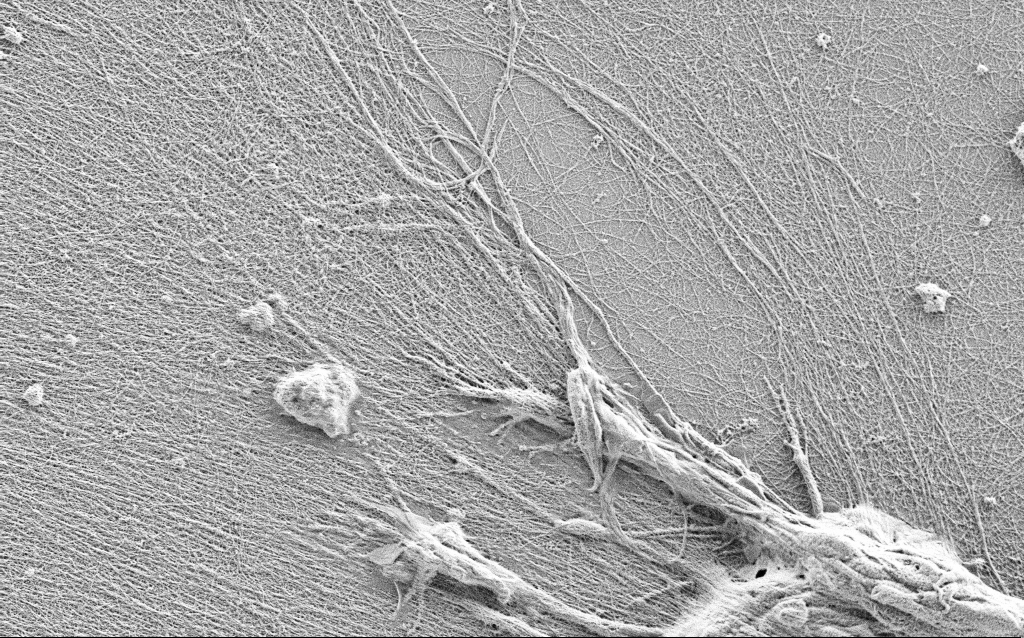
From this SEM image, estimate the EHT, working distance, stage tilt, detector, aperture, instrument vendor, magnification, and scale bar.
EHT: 0.9 kV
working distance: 3 mm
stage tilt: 0°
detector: SE2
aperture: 30 µm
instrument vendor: Zeiss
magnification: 10 K X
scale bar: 2000 nm